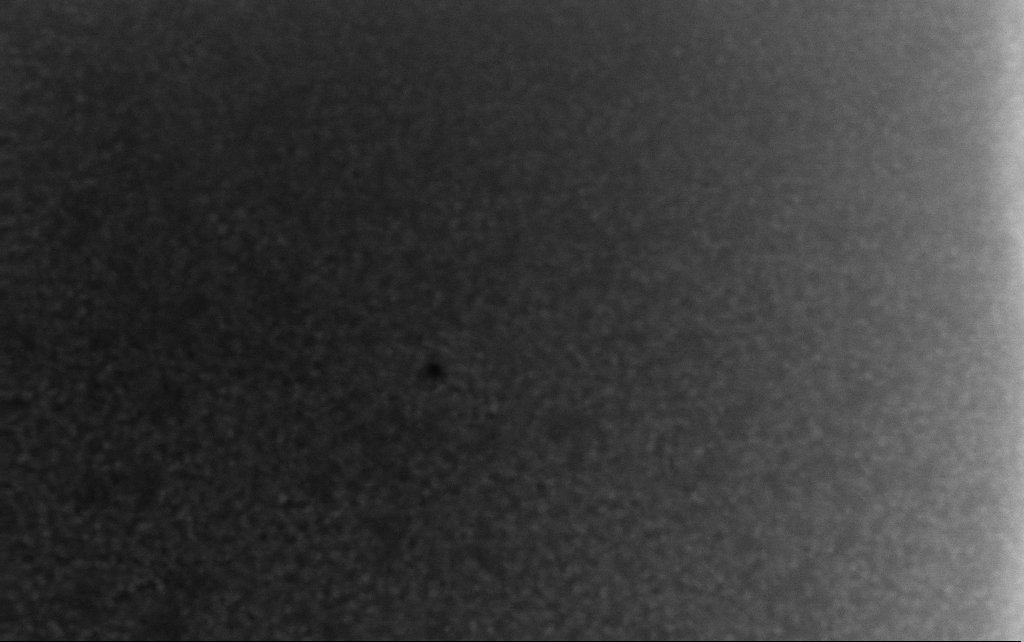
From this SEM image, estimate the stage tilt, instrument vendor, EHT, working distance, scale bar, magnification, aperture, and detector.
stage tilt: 0°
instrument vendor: Zeiss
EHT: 10 kV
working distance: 2.5 mm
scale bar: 100 nm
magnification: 329.69 K X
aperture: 30 µm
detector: InLens